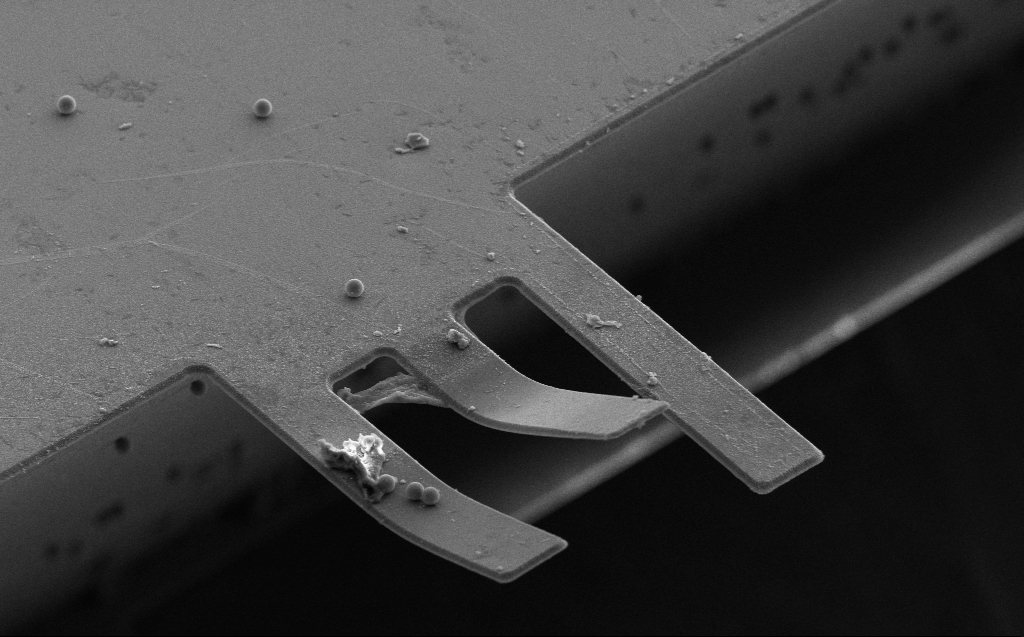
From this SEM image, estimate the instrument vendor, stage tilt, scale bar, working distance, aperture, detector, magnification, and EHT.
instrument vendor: Zeiss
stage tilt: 45.4°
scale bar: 20000 nm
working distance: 11 mm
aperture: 30 µm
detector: SE2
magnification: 1.46 K X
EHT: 10 kV